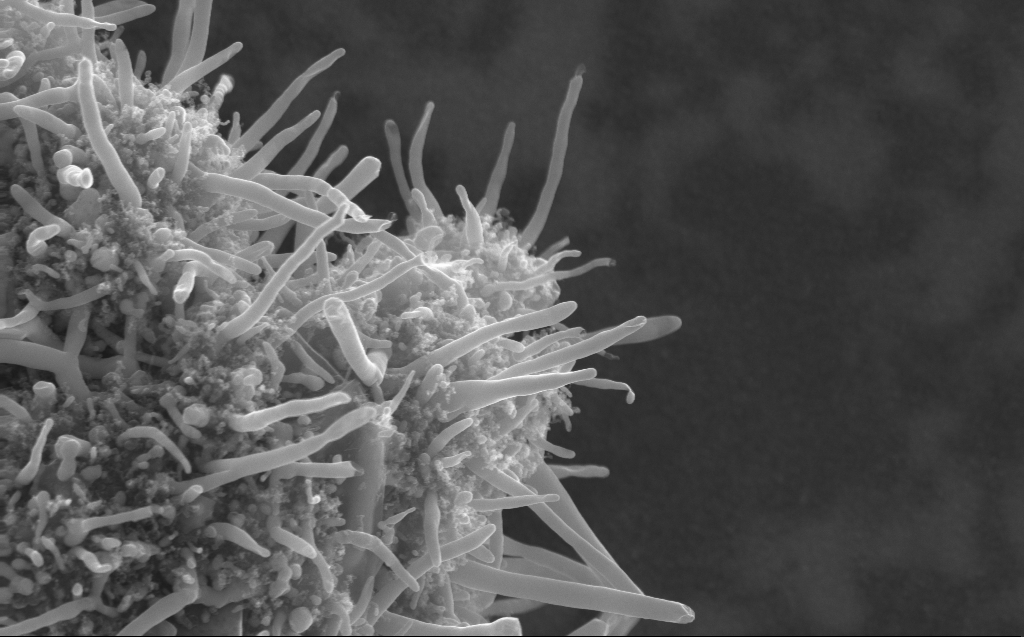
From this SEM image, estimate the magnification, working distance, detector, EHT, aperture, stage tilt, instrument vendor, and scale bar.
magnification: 52.16 K X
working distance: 3 mm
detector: InLens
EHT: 10 kV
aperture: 30 µm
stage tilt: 0°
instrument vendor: Zeiss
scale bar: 1000 nm